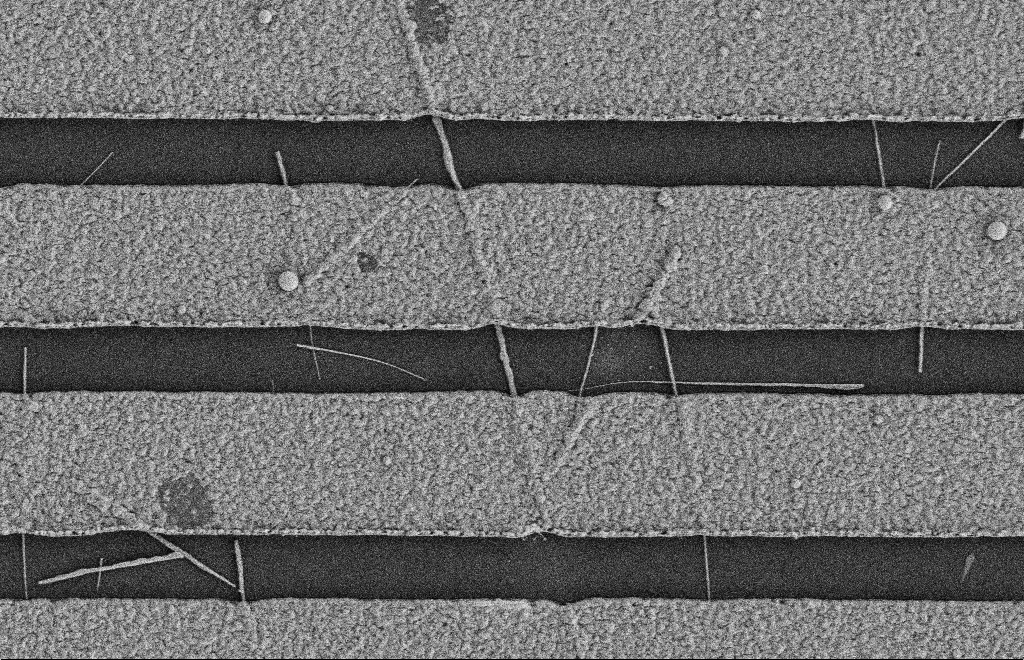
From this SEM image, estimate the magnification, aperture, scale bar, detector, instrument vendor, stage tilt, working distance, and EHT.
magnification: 19.08 K X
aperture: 20 µm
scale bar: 2000 nm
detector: SE2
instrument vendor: Zeiss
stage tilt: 0°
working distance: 9 mm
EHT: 2 kV